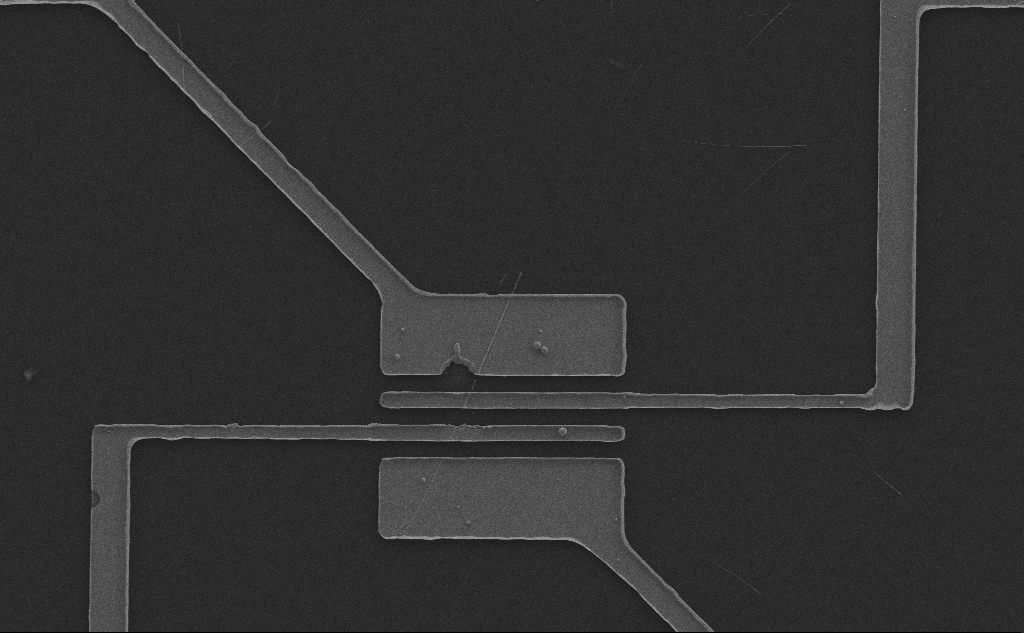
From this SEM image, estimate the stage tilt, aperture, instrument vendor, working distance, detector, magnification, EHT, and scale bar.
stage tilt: -0.1°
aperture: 30 µm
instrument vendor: Zeiss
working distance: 6 mm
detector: SE2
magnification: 3.04 K X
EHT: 10 kV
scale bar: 20000 nm